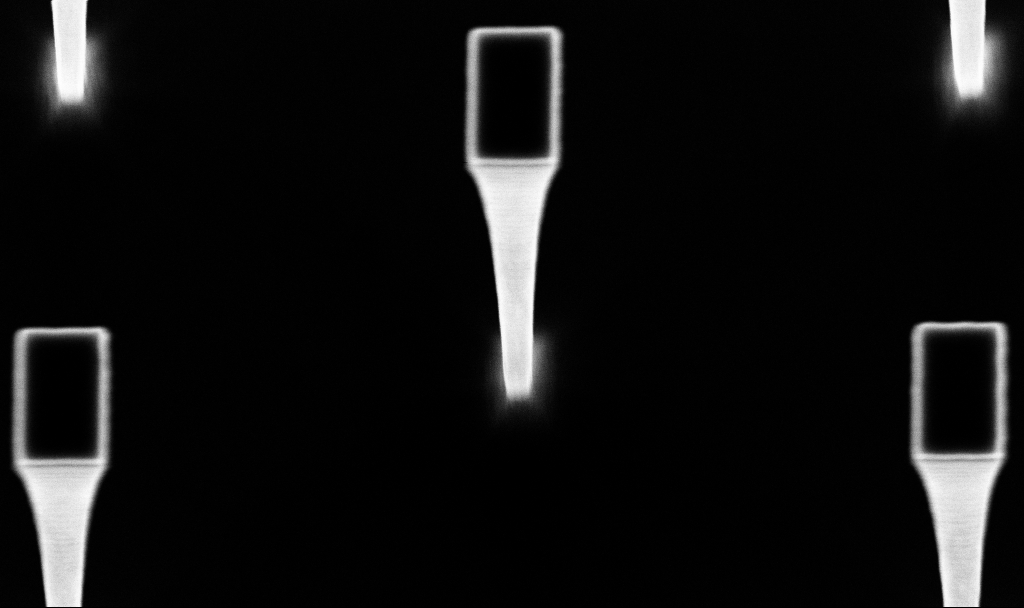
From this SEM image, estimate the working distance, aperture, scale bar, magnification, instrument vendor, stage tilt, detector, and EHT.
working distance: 4.8 mm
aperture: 30 µm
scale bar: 1000 nm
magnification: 23.14 K X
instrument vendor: Zeiss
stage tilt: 15°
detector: InLens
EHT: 5 kV